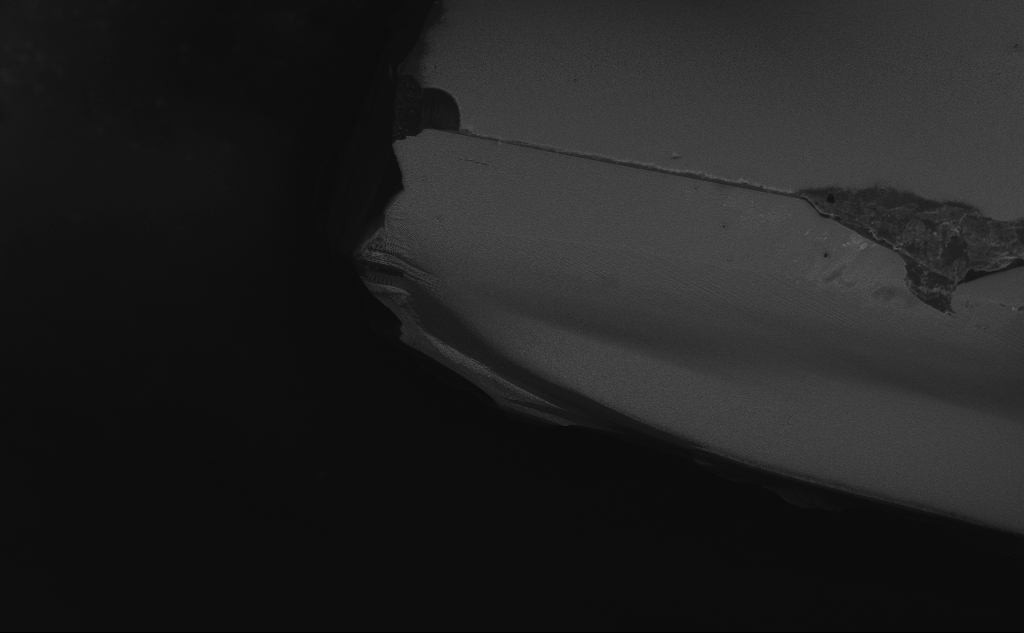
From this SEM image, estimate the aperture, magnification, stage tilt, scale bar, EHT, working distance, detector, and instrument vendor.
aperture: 30 µm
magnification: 0.298 K X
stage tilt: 45°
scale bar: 100000 nm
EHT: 10 kV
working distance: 6 mm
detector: InLens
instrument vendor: Zeiss